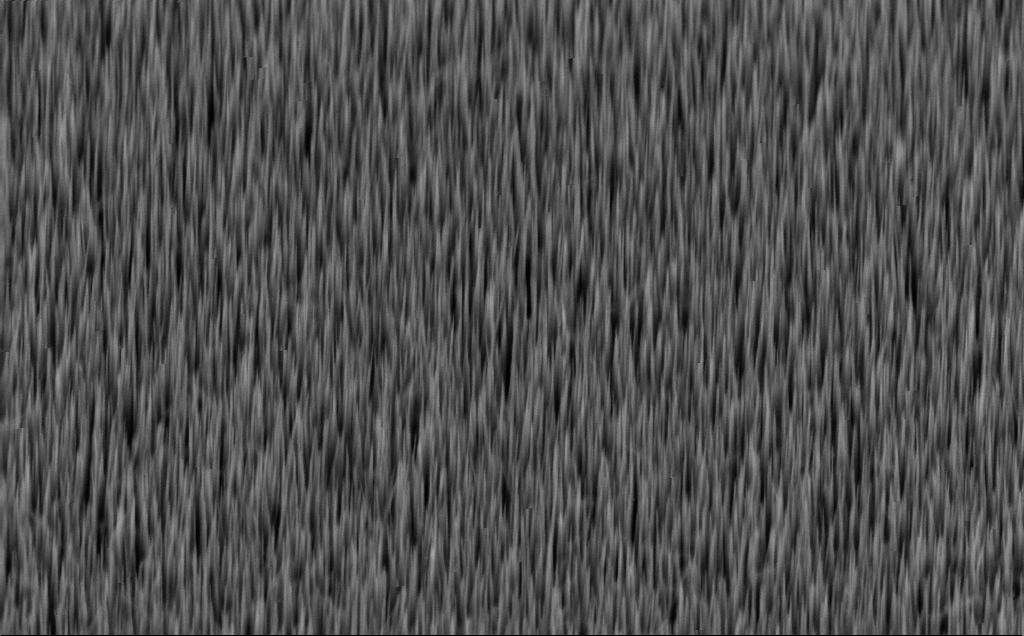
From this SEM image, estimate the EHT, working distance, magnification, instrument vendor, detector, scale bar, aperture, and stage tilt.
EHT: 10 kV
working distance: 5 mm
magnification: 40 K X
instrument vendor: Zeiss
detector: InLens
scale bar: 1000 nm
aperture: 30 µm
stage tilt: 45°